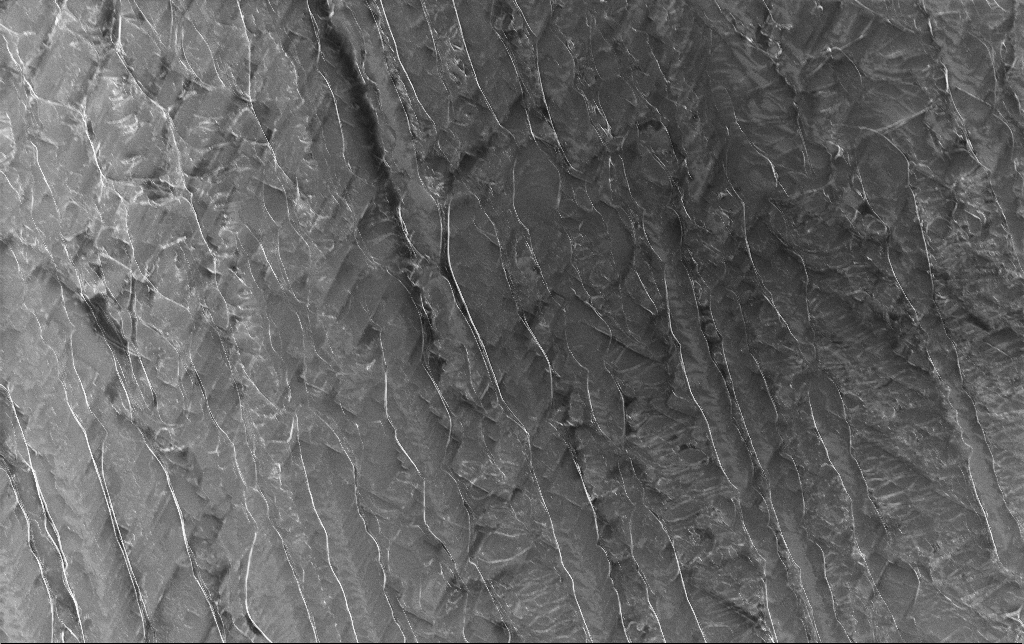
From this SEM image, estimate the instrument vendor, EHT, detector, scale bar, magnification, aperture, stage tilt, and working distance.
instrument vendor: Zeiss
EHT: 5 kV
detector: InLens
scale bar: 100000 nm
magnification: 0.63 K X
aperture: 30 µm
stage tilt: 0°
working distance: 3 mm